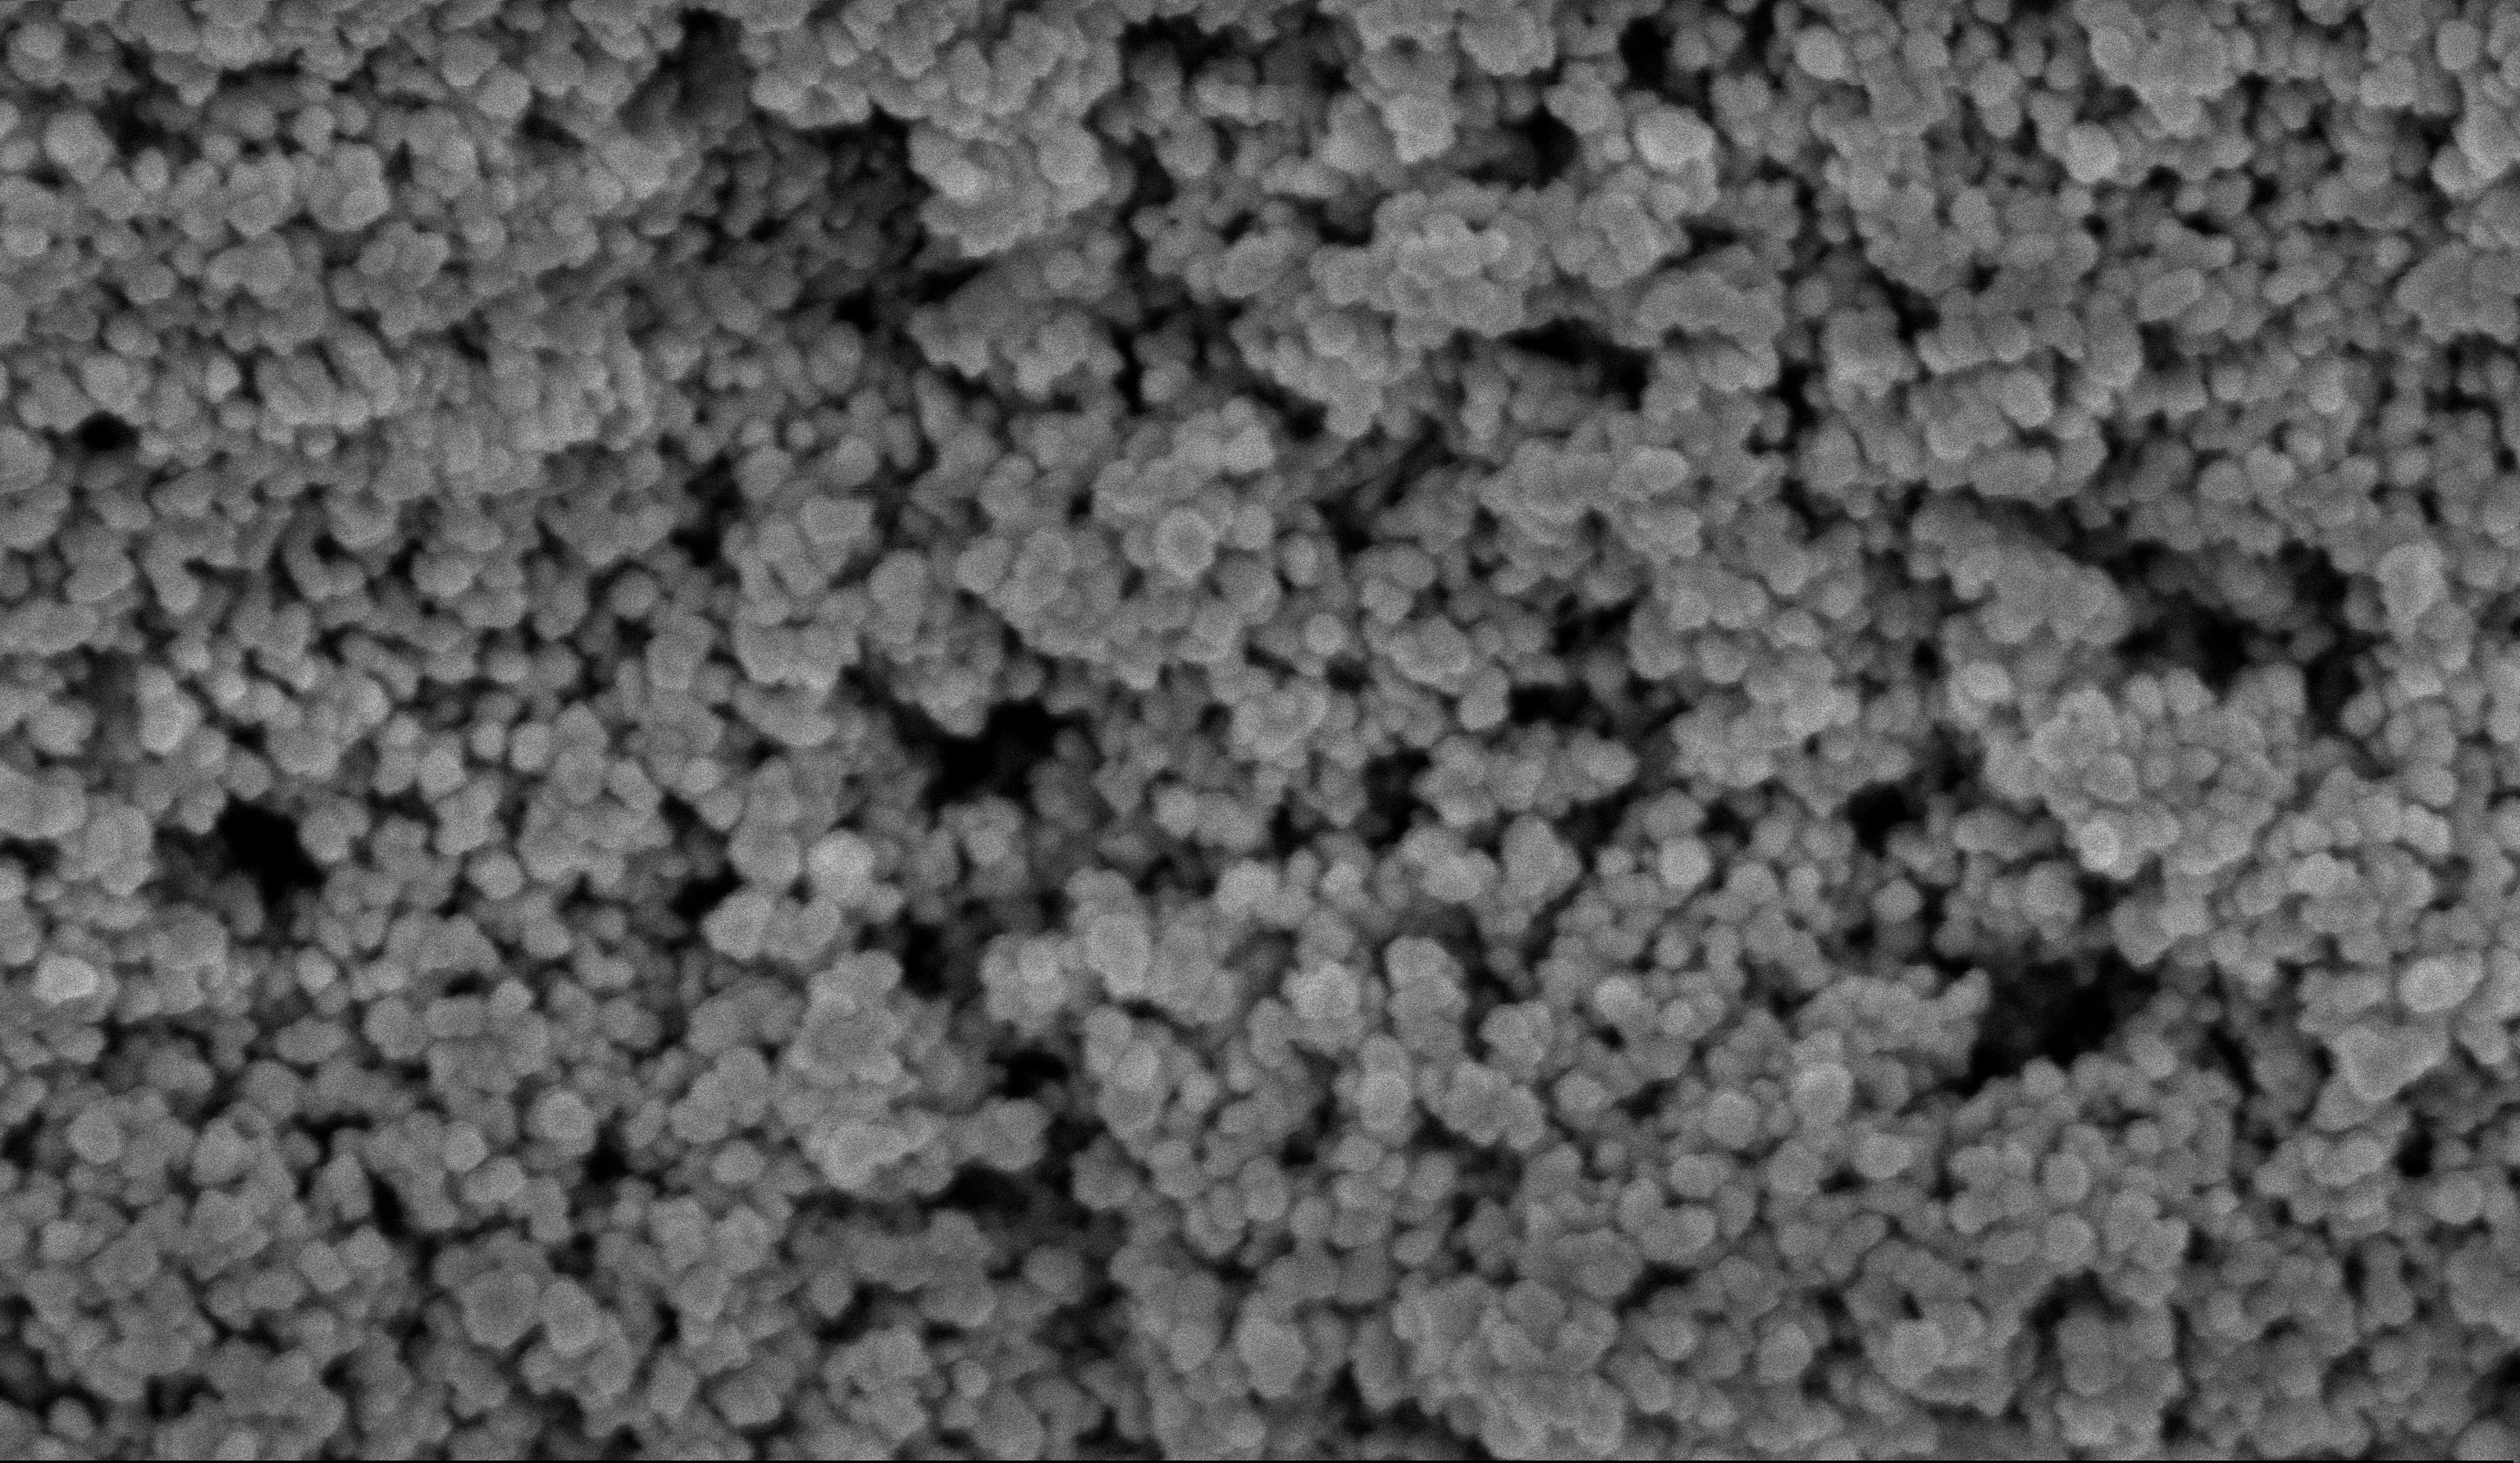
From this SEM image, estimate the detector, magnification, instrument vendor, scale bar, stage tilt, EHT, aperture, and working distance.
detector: InLens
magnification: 135 K X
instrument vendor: Zeiss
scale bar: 200 nm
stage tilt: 0°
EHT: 5 kV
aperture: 30 µm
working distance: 5.9 mm